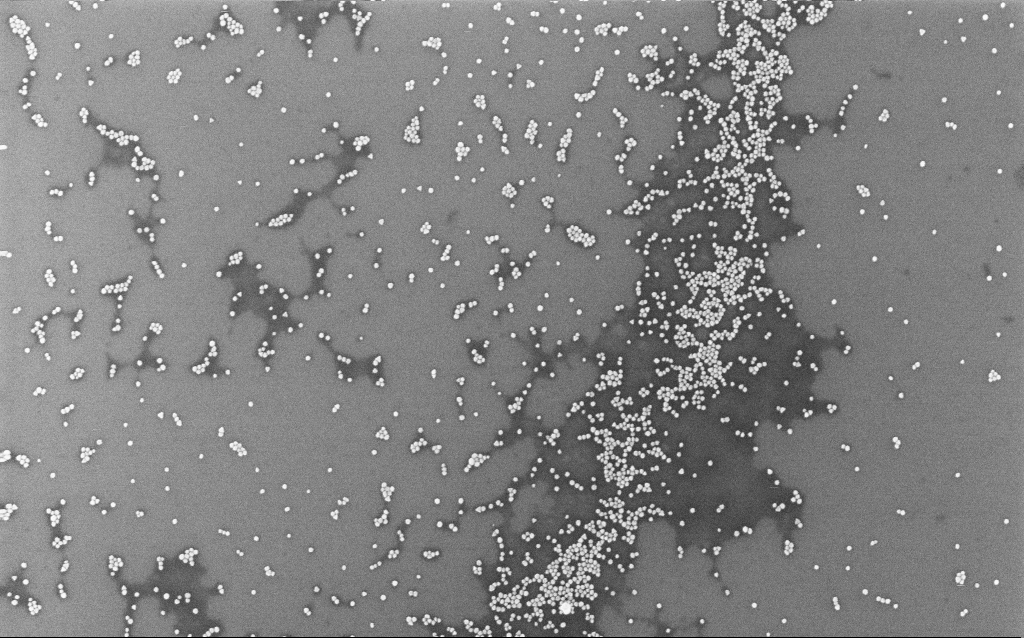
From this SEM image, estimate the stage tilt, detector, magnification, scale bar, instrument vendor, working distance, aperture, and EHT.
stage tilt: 0°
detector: InLens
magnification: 100 K X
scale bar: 200 nm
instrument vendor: Zeiss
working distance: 1.9 mm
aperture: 30 µm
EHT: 10 kV